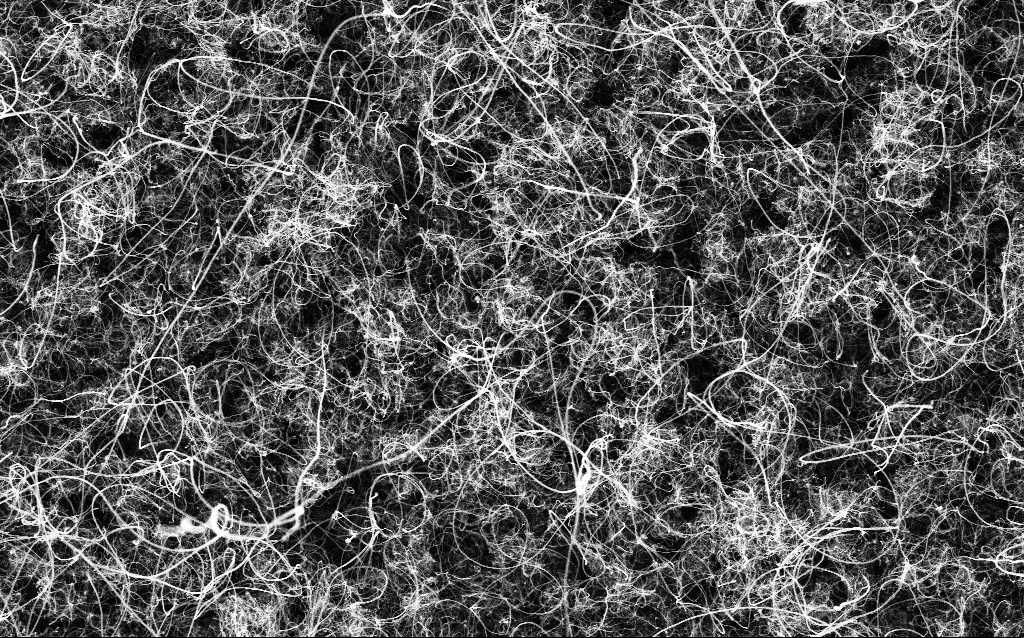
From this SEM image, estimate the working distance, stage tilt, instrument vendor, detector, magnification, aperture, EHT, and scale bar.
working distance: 3.6 mm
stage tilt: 0°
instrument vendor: Zeiss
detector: InLens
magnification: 20 K X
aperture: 30 µm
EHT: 5 kV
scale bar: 1000 nm